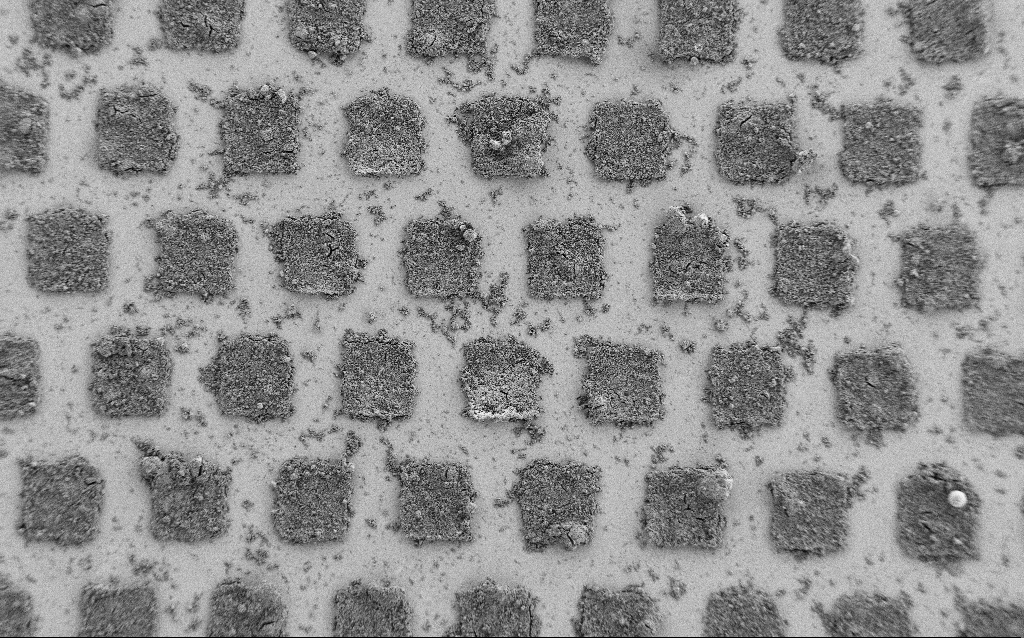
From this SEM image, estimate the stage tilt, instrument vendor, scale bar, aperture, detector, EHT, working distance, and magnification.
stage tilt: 0°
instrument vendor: Zeiss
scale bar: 100000 nm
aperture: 30 µm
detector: SE2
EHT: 1 kV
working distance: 5.6 mm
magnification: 0.449 K X